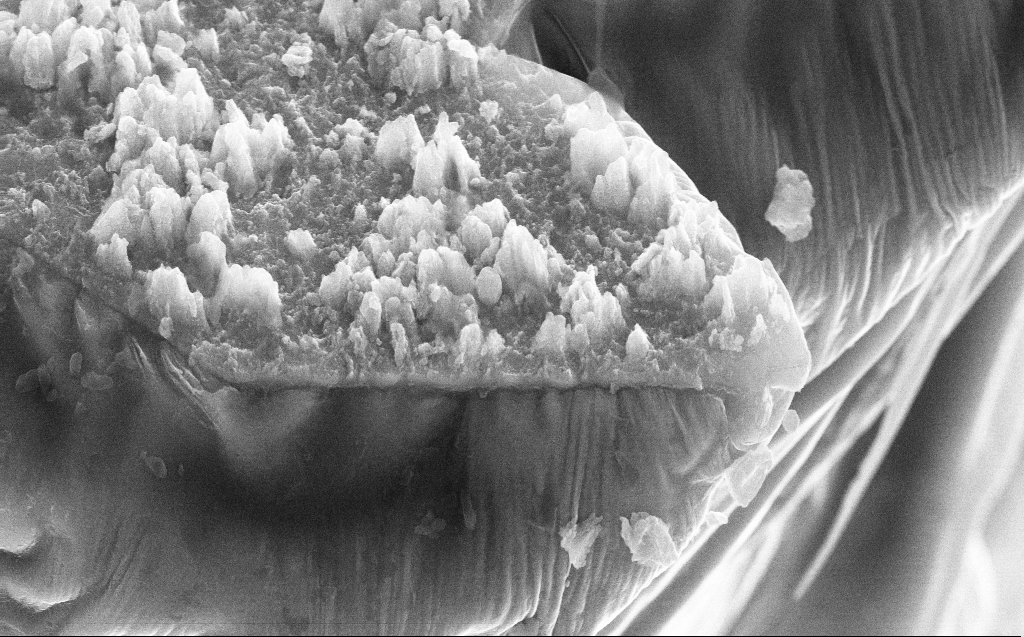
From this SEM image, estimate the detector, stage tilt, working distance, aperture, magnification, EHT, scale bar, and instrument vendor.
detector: SE2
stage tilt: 45°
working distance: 8 mm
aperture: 30 µm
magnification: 18.51 K X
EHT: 30 kV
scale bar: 1000 nm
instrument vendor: Zeiss